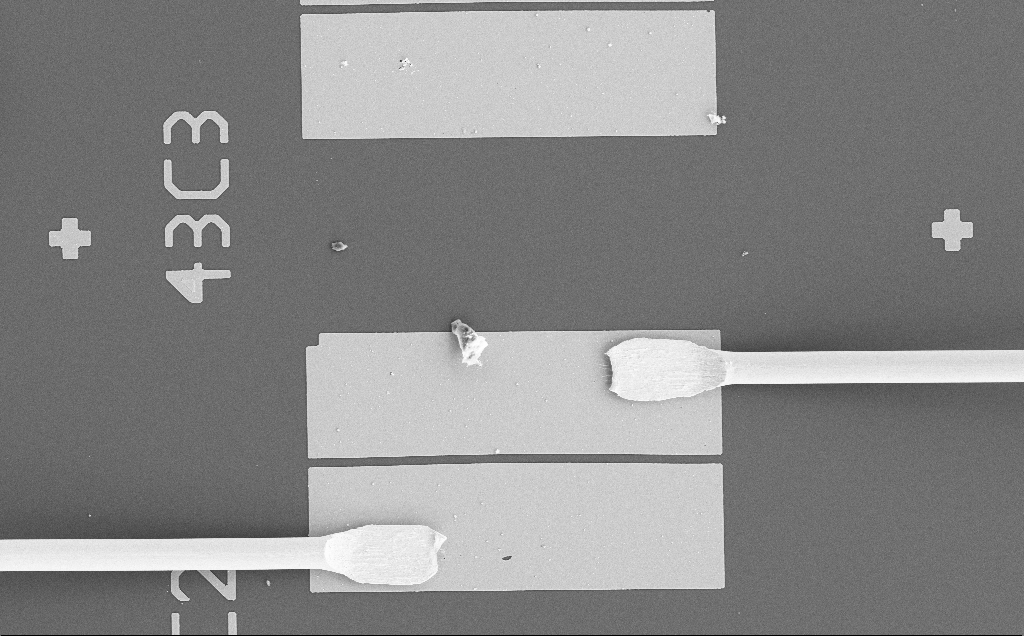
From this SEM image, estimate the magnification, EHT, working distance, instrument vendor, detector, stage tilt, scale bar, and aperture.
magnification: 0.476 K X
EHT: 15 kV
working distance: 11 mm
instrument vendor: Zeiss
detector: SE2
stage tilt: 0°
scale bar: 100000 nm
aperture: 30 µm